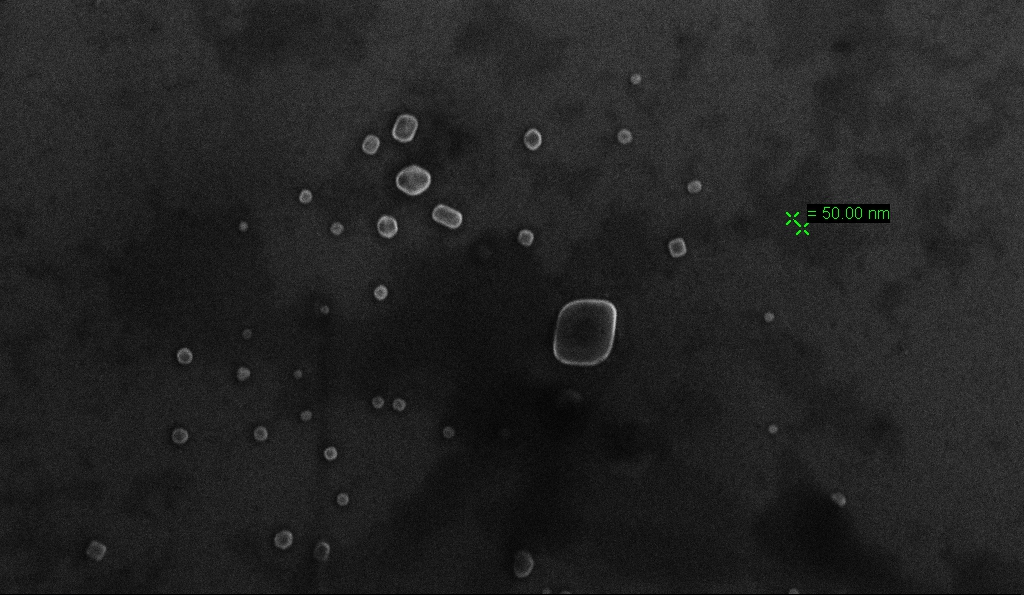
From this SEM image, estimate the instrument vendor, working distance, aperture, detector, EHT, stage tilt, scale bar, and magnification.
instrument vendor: Zeiss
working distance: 5.3 mm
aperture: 30 µm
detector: InLens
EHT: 10 kV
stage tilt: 0°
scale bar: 200 nm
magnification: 108.63 K X